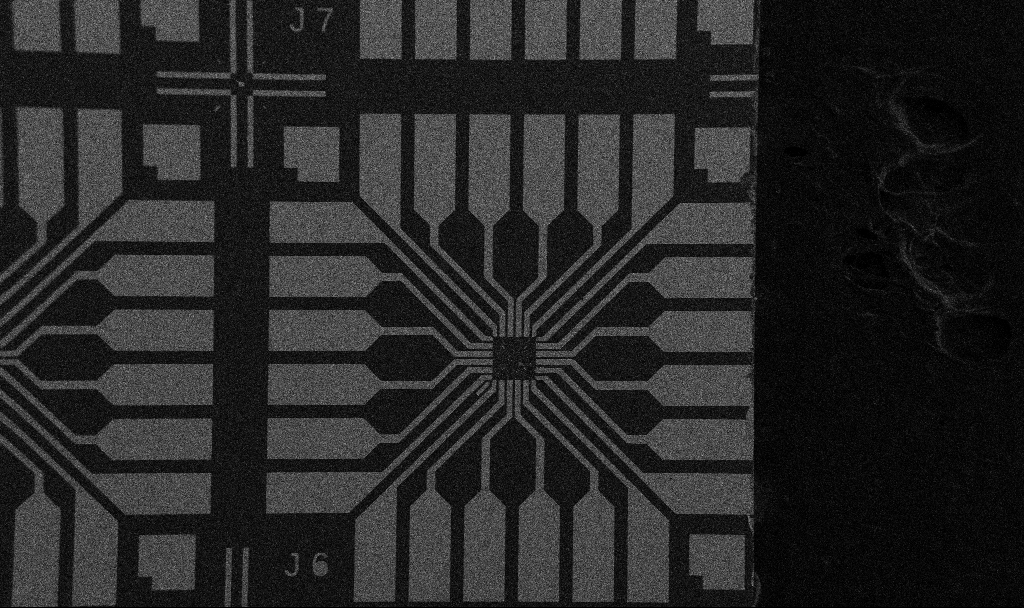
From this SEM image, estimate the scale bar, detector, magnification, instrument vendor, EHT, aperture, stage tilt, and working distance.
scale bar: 200000 nm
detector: SE2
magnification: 0.1 K X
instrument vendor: Zeiss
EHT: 5 kV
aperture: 30 µm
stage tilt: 0°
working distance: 10.7 mm